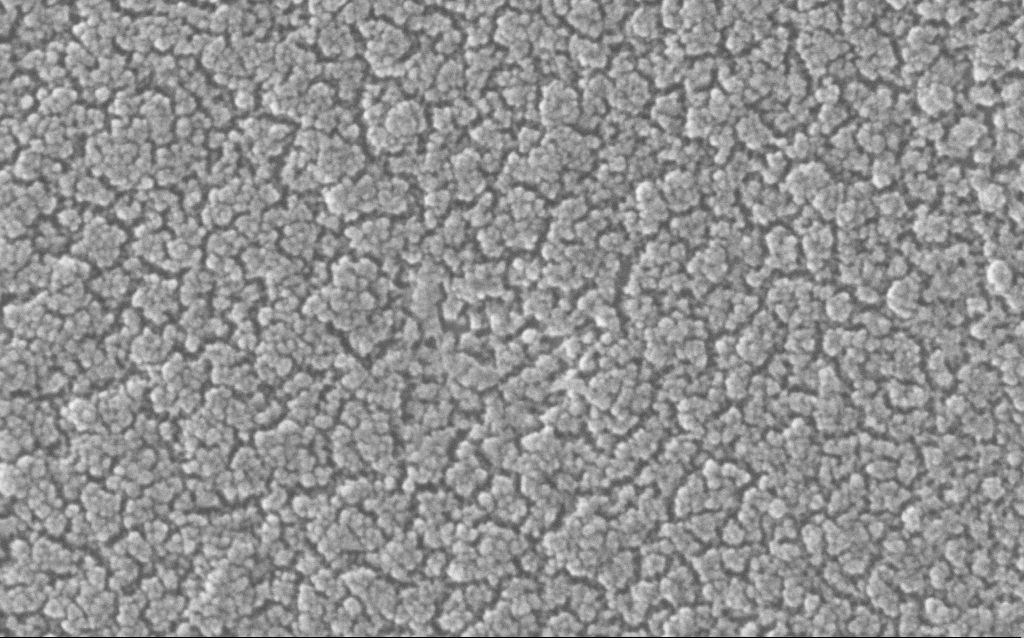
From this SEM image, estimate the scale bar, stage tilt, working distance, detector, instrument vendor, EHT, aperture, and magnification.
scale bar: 100 nm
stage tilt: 0°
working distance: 1.8 mm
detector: InLens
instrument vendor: Zeiss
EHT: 20 kV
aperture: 30 µm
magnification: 500 K X